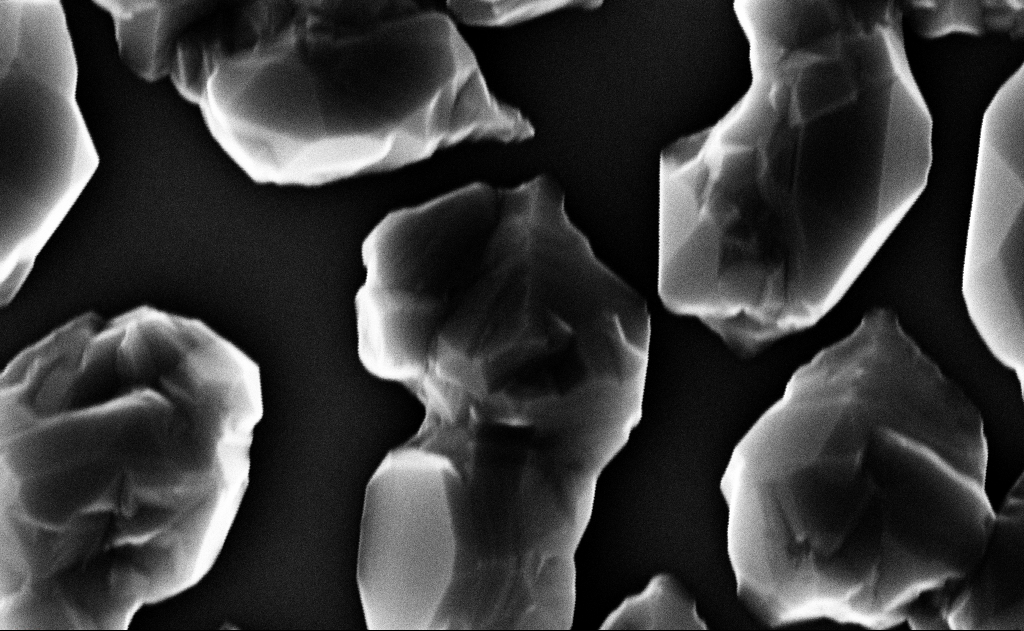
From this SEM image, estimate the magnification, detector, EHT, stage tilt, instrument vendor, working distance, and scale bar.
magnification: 100 K X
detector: InLens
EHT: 10 kV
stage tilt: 0°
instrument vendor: Zeiss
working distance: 12 mm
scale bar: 200 nm